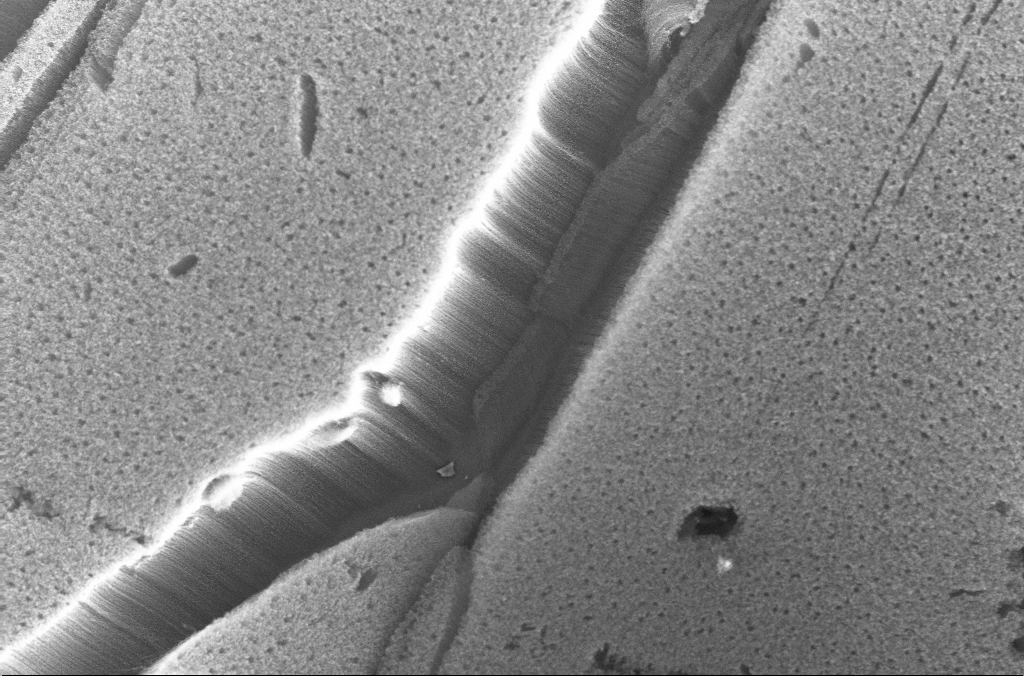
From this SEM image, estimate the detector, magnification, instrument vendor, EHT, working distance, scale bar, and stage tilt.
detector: InLens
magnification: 2 K X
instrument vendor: Zeiss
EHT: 10 kV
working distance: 4 mm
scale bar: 20000 nm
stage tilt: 0°